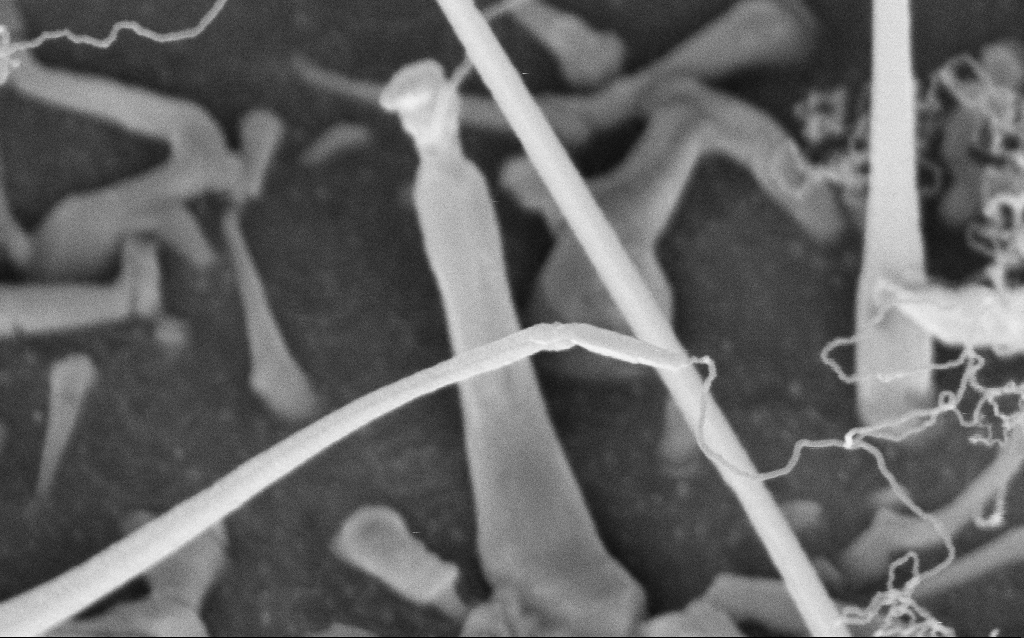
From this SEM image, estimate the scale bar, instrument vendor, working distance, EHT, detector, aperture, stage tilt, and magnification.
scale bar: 200 nm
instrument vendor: Zeiss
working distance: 8 mm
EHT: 5 kV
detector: InLens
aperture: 30 µm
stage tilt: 42°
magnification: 150.84 K X